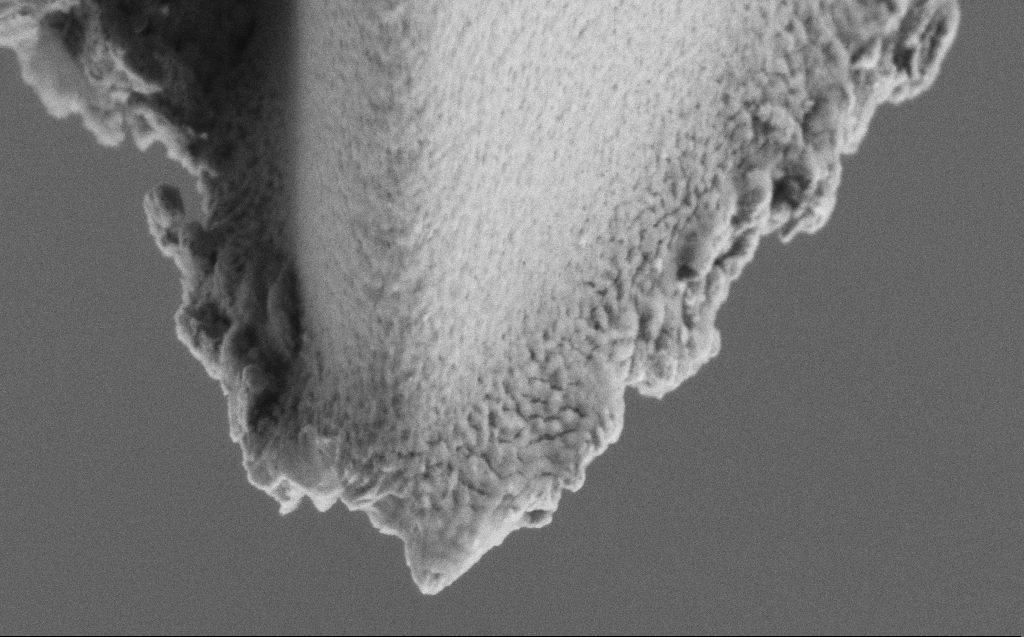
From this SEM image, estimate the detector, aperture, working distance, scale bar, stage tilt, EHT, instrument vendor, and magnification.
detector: SE2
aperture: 30 µm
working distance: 4 mm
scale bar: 1000 nm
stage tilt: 45°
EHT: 3 kV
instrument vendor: Zeiss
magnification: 48.2 K X